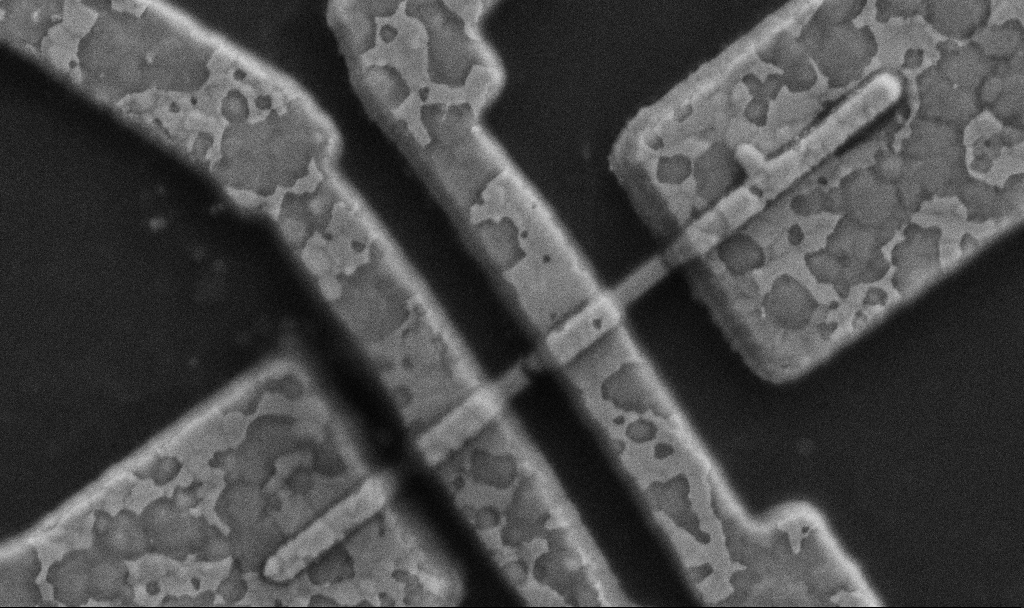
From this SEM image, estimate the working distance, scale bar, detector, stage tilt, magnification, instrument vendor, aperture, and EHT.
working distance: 8.5 mm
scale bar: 1000 nm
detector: SE2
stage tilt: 0°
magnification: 50 K X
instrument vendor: Zeiss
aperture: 30 µm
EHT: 5 kV